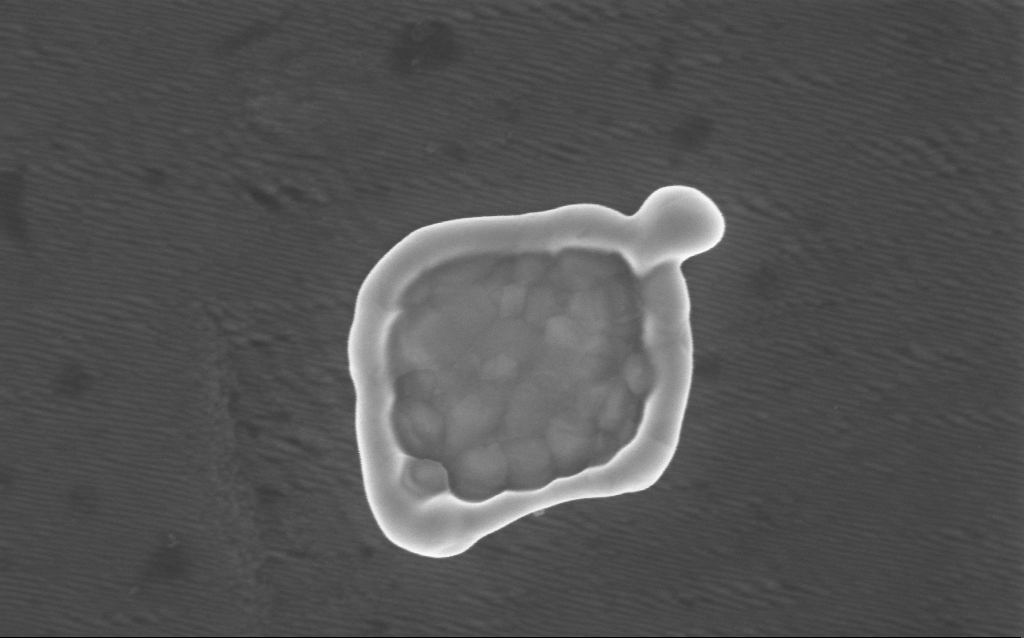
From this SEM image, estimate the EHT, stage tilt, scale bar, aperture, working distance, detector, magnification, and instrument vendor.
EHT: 5 kV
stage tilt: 0°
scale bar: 200 nm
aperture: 30 µm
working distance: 5 mm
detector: InLens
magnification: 144.38 K X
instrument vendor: Zeiss